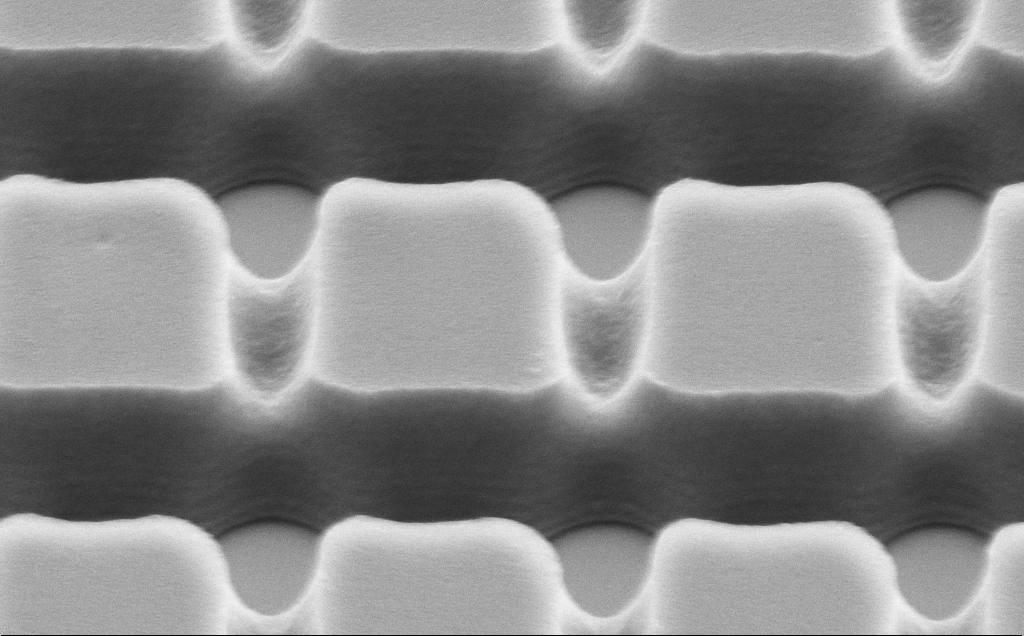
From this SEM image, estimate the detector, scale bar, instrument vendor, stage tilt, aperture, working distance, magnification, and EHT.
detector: SE2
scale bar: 2000 nm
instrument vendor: Zeiss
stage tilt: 45°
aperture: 30 µm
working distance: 10 mm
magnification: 30.56 K X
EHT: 5 kV